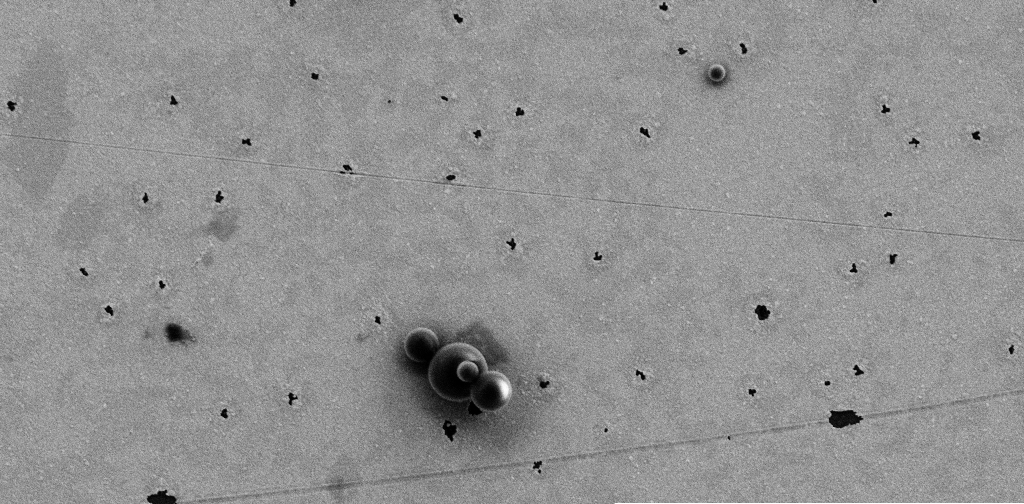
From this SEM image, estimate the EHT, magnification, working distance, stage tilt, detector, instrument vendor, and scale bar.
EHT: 3 kV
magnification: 13.37 K X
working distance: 10 mm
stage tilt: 0°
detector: SE2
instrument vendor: Zeiss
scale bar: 2000 nm